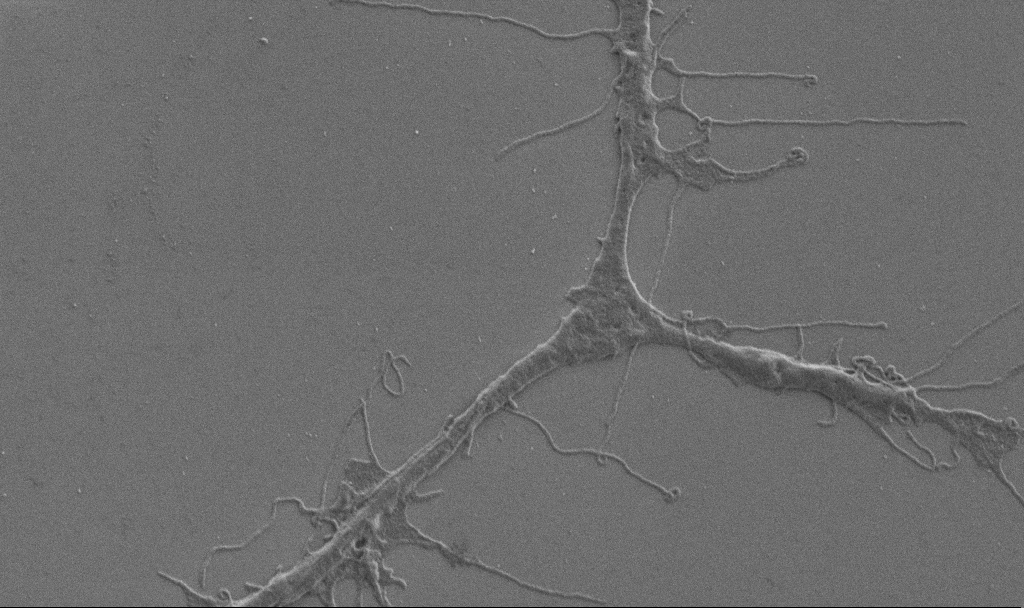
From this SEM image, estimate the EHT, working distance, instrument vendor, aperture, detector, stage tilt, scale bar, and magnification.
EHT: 1 kV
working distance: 6.9 mm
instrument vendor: Zeiss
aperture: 30 µm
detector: SE2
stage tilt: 0°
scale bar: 2000 nm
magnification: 10 K X